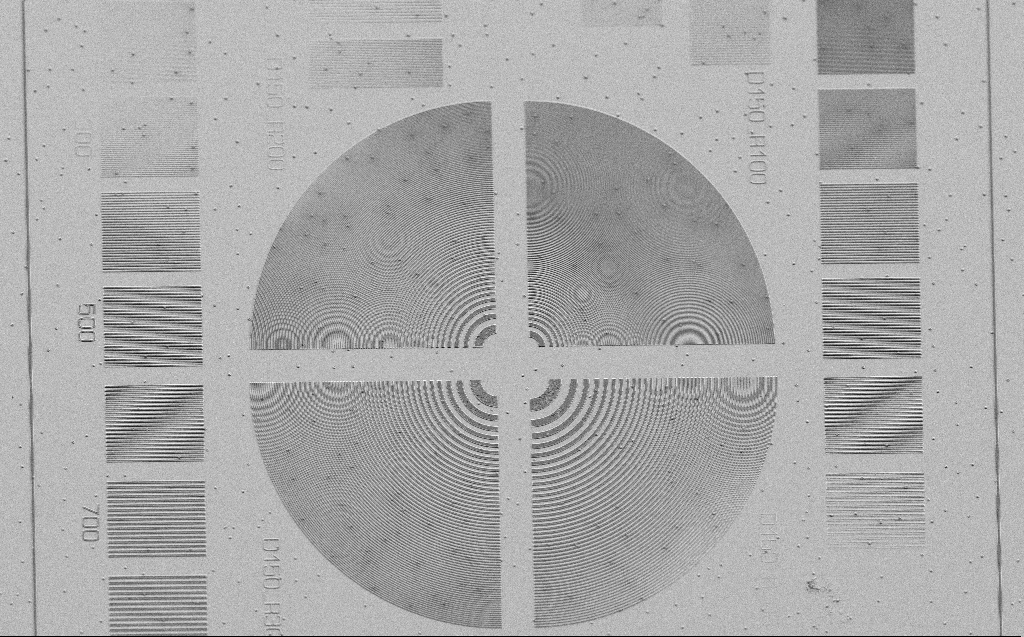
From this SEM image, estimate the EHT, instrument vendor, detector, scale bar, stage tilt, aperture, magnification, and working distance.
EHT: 3 kV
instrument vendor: Zeiss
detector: SE2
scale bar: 10000 nm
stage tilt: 30°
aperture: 30 µm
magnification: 1.21 K X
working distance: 6 mm